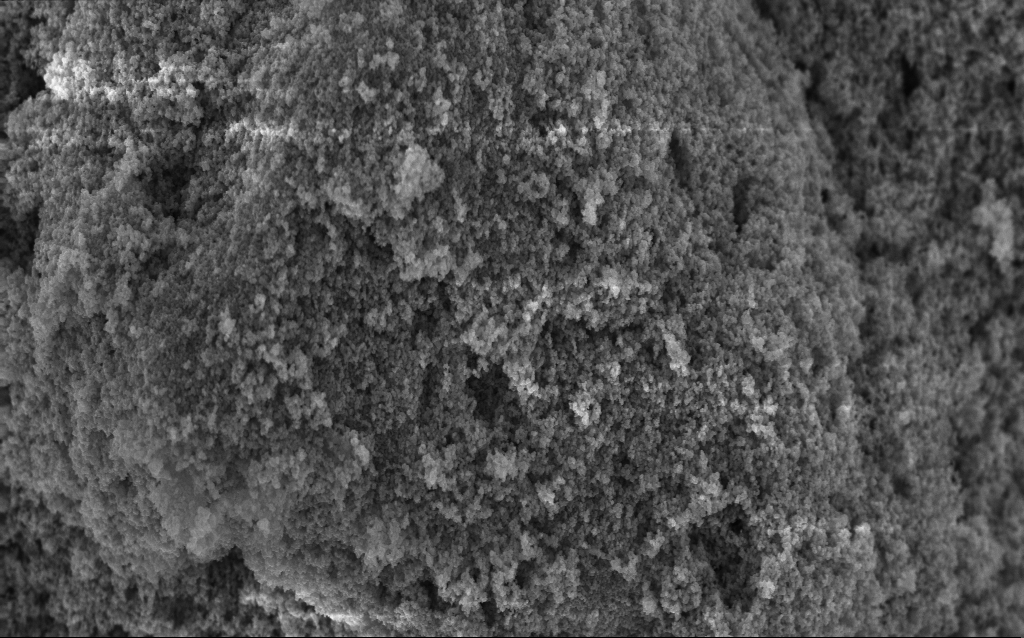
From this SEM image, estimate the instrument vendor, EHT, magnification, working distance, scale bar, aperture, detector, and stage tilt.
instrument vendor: Zeiss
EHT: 5 kV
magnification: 37.8 K X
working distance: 2.5 mm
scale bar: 1000 nm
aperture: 30 µm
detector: InLens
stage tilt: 0°